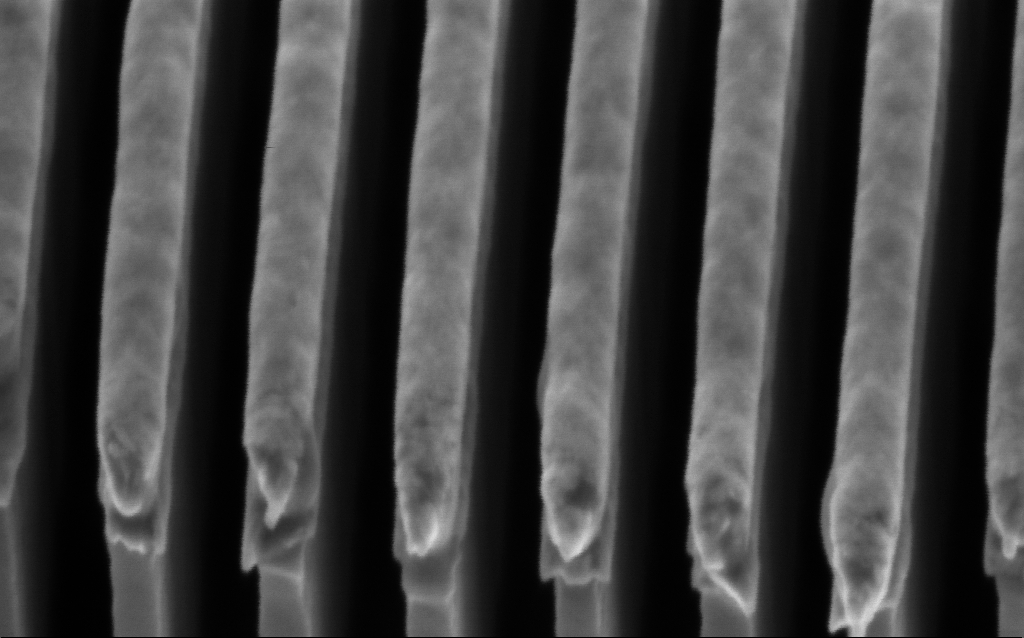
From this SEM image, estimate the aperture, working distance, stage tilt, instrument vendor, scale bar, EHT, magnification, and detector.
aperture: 30 µm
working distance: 3.2 mm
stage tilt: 45°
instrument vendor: Zeiss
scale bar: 200 nm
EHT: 2 kV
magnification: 299.05 K X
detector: InLens